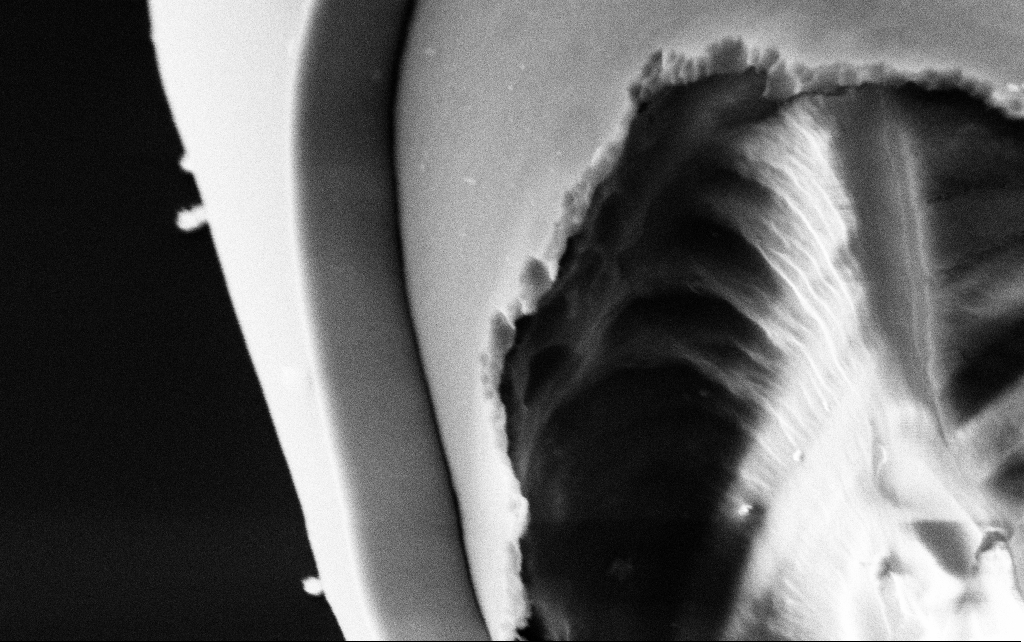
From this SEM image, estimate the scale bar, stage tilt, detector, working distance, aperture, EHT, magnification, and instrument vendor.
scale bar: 200 nm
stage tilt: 45°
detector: SE2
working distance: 7.9 mm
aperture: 30 µm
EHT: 3 kV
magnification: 75 K X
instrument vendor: Zeiss